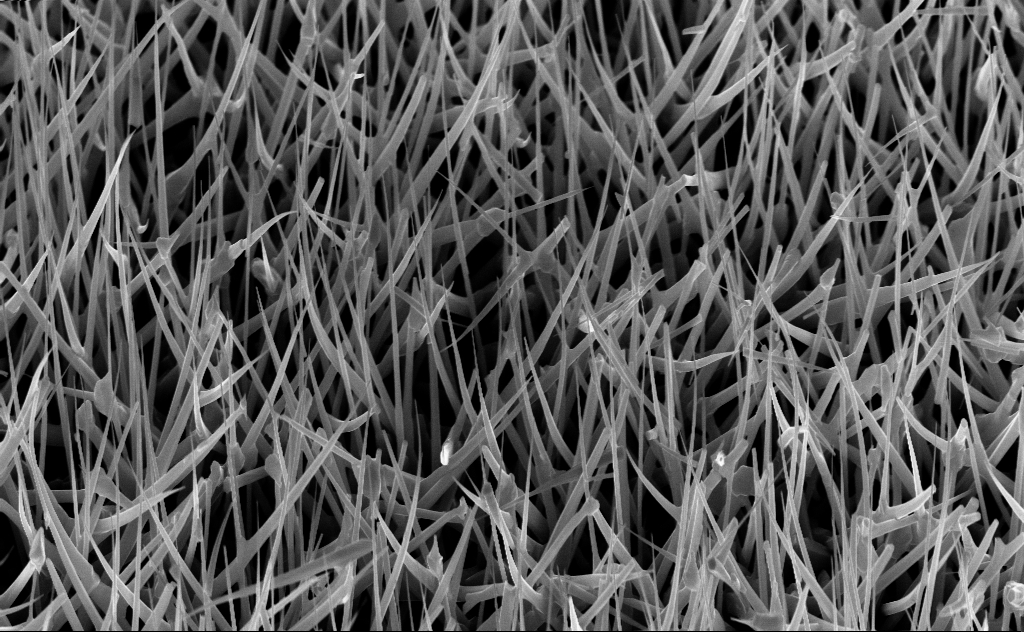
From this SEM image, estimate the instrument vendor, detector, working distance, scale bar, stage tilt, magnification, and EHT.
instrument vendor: Zeiss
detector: InLens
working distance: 7 mm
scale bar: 2000 nm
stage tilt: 45°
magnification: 20 K X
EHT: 10 kV